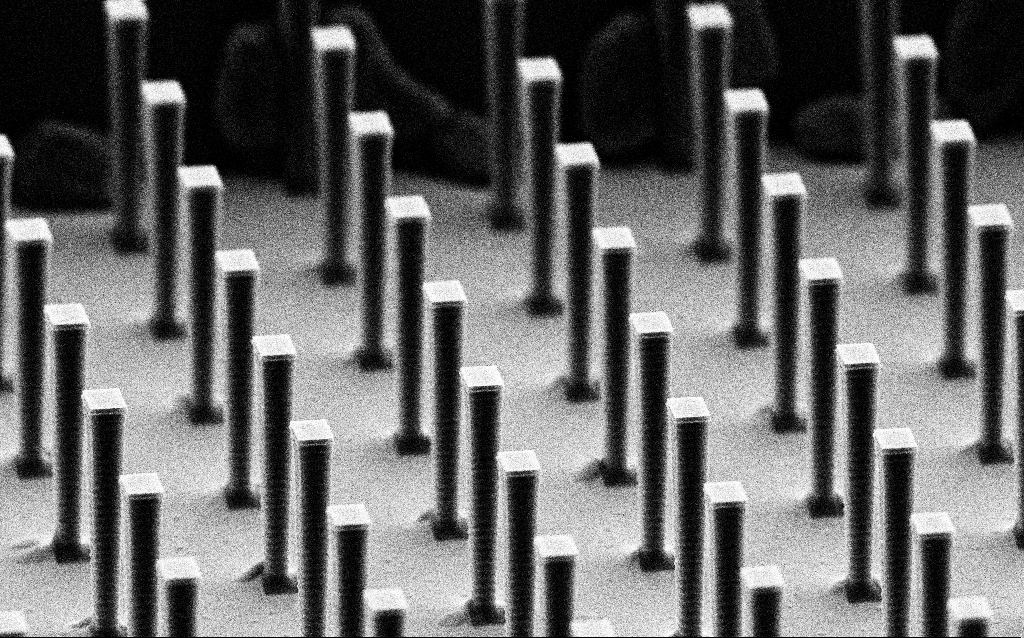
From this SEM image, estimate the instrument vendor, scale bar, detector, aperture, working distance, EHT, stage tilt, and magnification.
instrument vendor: Zeiss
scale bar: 2000 nm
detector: SE2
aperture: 30 µm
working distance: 6.5 mm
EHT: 8 kV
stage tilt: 70°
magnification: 7.74 K X